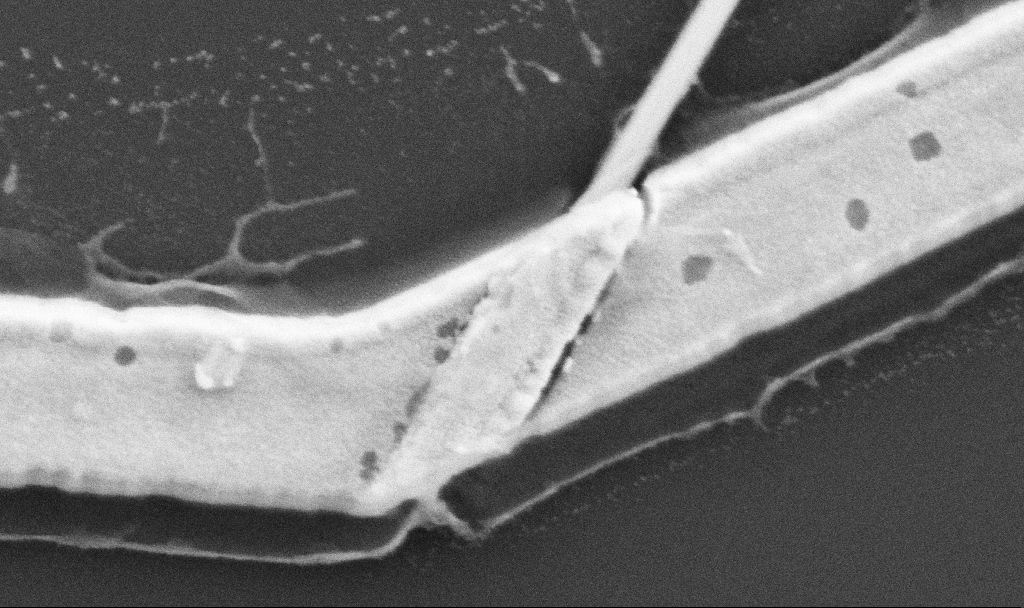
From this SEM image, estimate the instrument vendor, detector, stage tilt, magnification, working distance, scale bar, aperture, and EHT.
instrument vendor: Zeiss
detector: SE2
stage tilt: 0°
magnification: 100 K X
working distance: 10.7 mm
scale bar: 200 nm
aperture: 30 µm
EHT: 5 kV